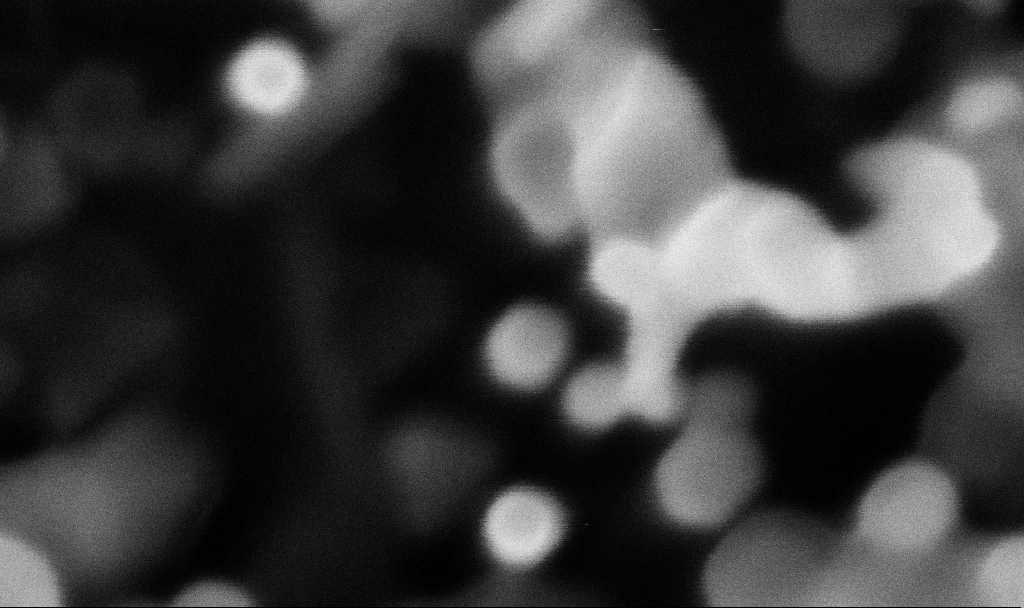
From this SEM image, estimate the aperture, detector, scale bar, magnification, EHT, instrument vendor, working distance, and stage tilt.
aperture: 30 µm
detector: InLens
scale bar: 100 nm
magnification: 700.58 K X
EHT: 3 kV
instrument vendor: Zeiss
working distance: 3.1 mm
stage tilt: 0°